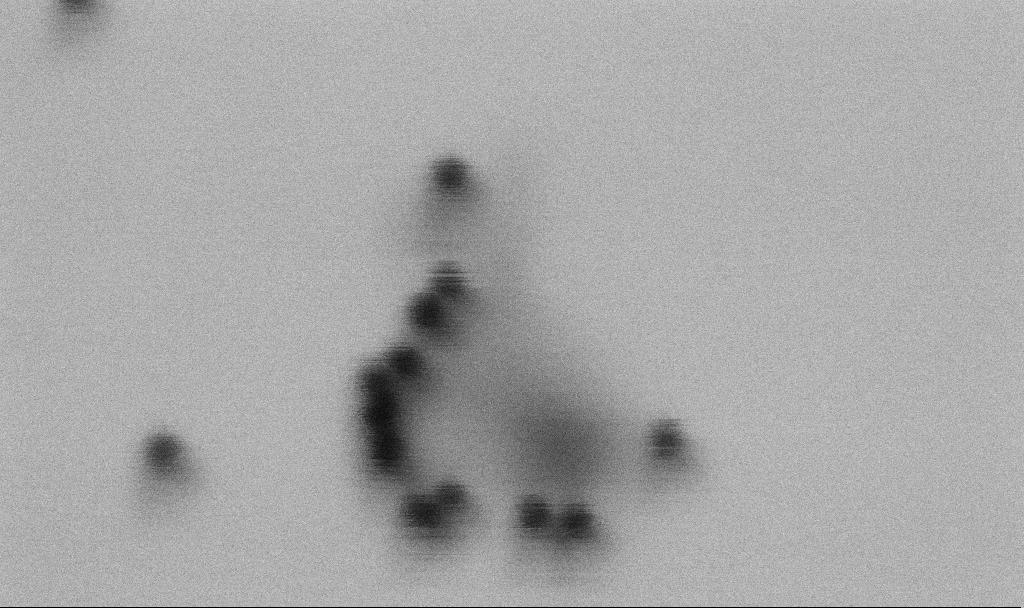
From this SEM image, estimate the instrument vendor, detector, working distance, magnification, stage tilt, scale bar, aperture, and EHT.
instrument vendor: Zeiss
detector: SE2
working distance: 6.5 mm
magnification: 600 K X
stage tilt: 0°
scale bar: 100 nm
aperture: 30 µm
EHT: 2 kV